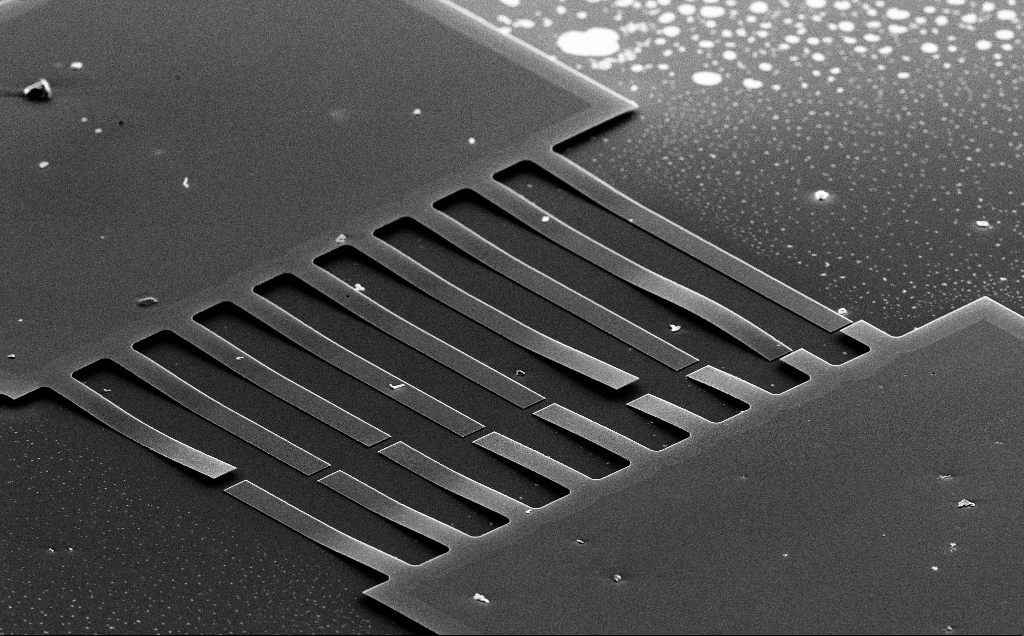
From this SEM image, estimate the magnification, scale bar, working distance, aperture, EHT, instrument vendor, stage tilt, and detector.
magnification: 0.742 K X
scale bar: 20000 nm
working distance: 14 mm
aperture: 30 µm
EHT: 10 kV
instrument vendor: Zeiss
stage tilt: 59.7°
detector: SE2